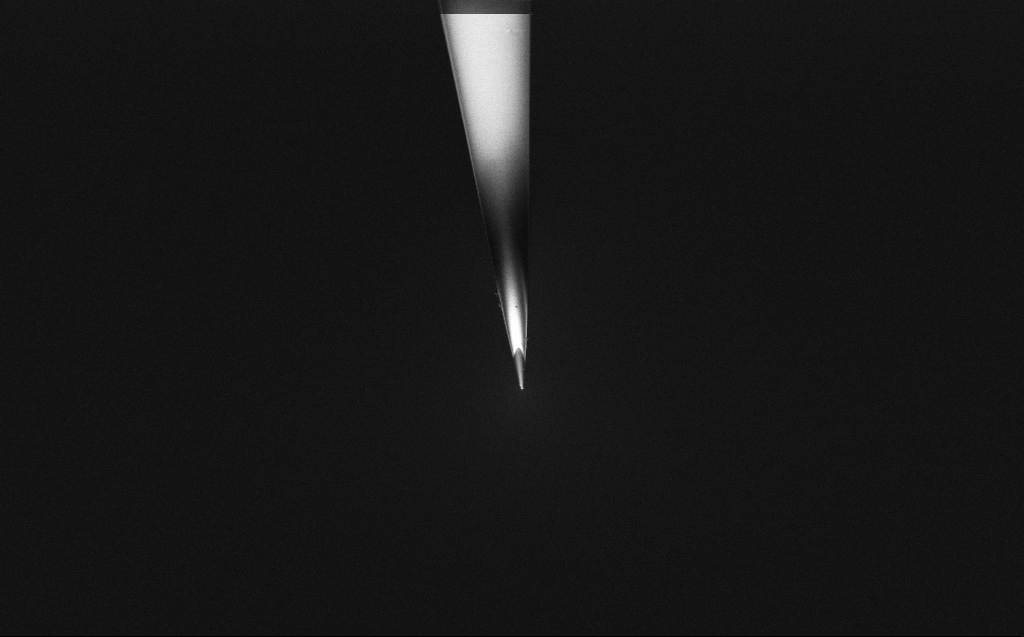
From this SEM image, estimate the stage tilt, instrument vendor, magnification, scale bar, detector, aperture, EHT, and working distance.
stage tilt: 45°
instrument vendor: Zeiss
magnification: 5 K X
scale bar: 10000 nm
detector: InLens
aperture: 30 µm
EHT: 2 kV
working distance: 6 mm